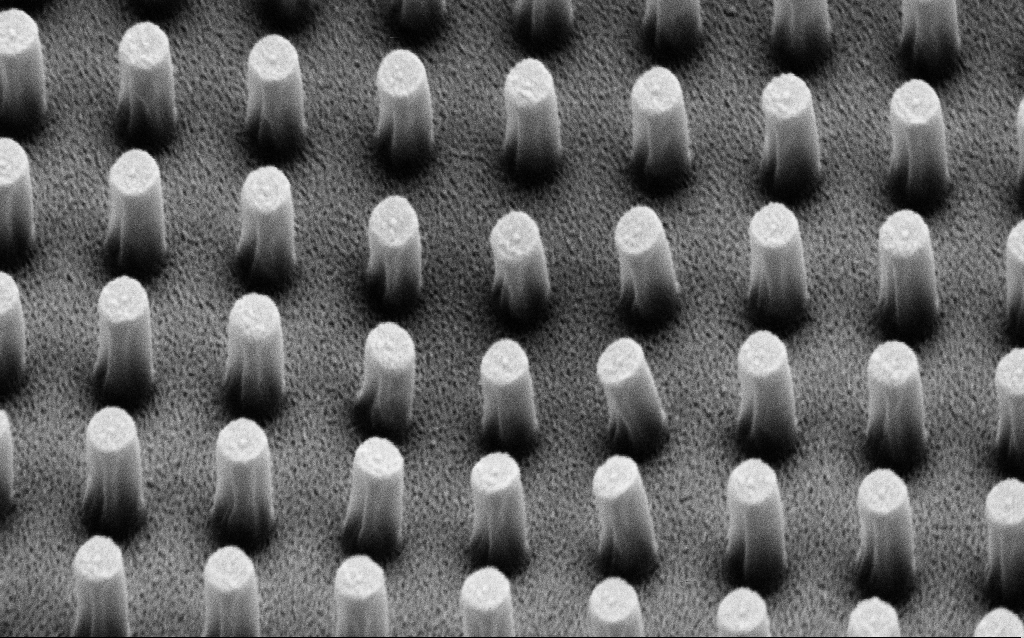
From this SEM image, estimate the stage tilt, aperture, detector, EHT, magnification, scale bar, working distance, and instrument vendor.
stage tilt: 45°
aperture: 30 µm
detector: SE2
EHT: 3 kV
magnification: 95.74 K X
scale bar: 200 nm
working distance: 6.7 mm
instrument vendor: Zeiss